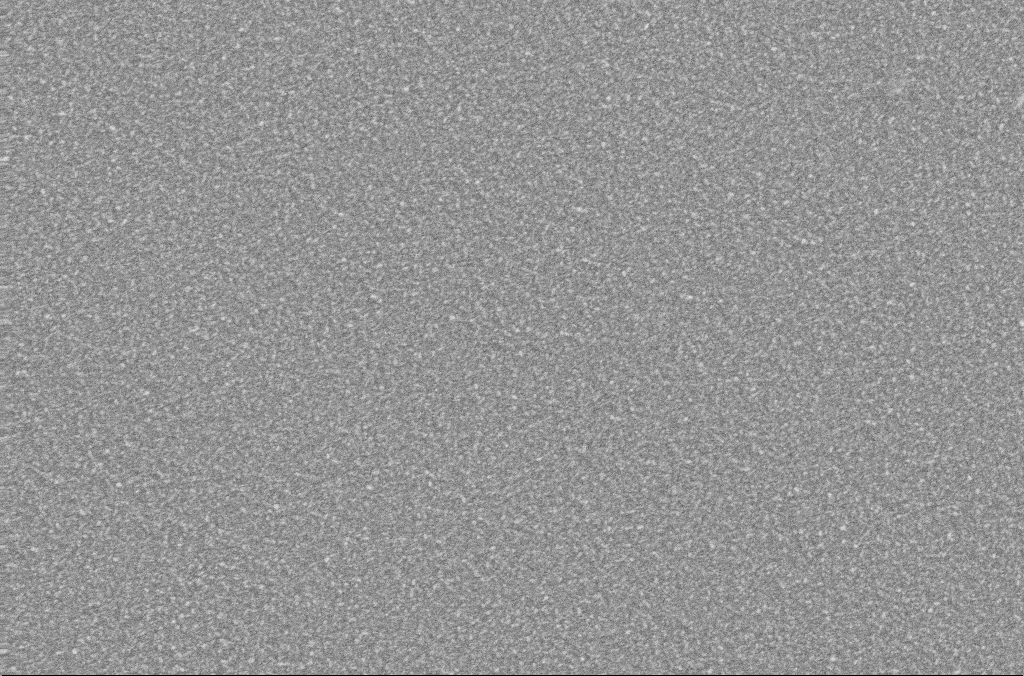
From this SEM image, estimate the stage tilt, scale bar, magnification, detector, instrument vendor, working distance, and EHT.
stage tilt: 0°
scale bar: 10000 nm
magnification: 5 K X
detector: SE2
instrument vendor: Zeiss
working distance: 2 mm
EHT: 20 kV